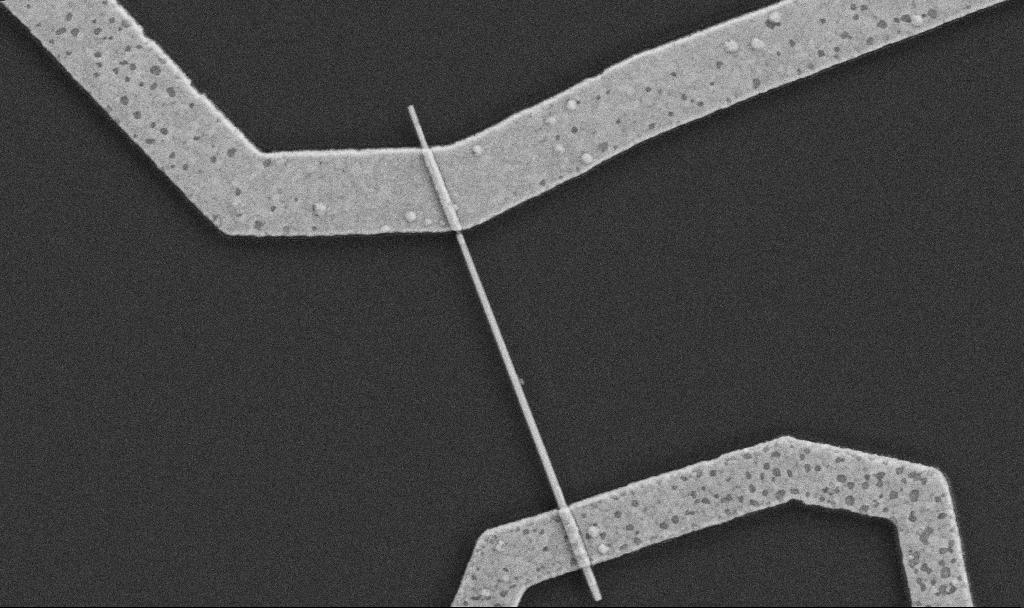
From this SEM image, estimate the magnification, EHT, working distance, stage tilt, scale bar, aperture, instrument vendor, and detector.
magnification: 30 K X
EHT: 5 kV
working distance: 7.6 mm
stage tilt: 0°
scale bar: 1000 nm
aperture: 30 µm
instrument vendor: Zeiss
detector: SE2